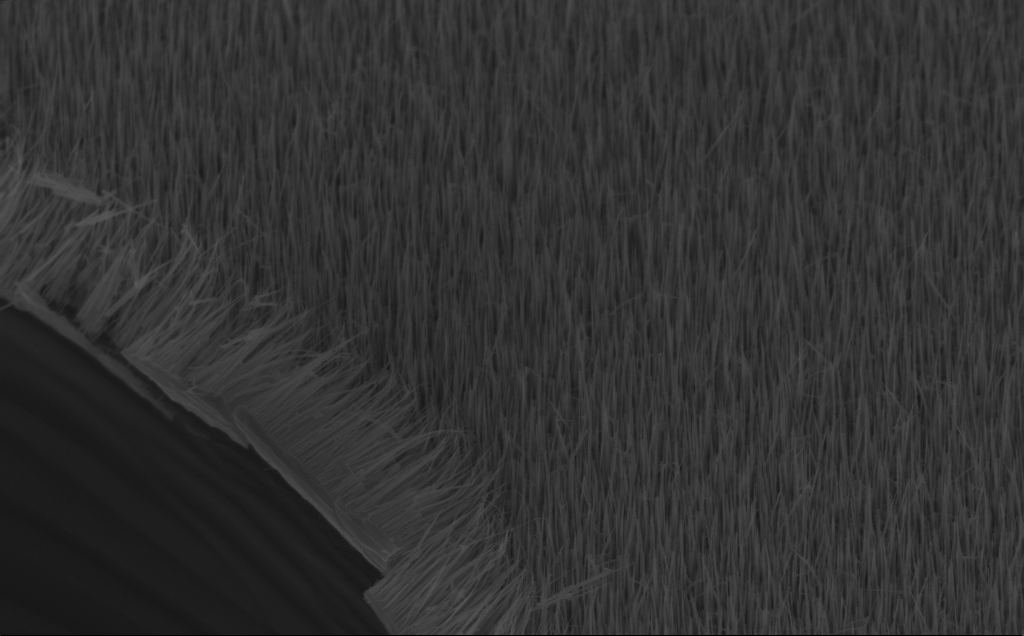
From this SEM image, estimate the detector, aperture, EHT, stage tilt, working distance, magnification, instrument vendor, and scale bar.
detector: InLens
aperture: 30 µm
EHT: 10 kV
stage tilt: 45°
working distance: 8 mm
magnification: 20 K X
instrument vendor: Zeiss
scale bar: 1000 nm